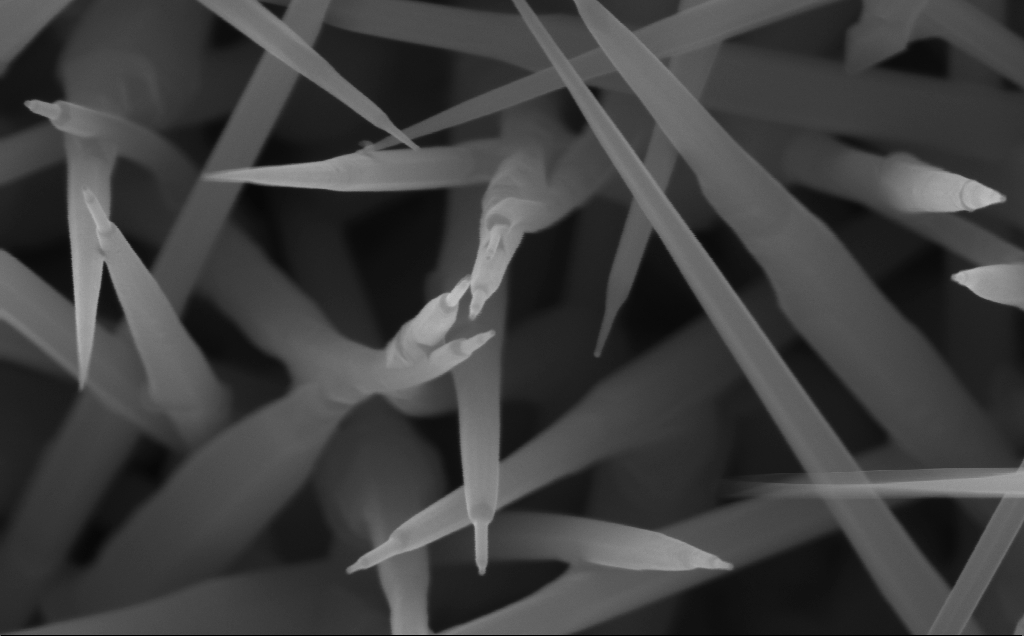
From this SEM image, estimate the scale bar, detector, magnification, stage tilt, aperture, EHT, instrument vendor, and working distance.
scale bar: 100 nm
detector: InLens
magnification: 150 K X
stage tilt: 0°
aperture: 30 µm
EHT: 10 kV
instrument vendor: Zeiss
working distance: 5 mm